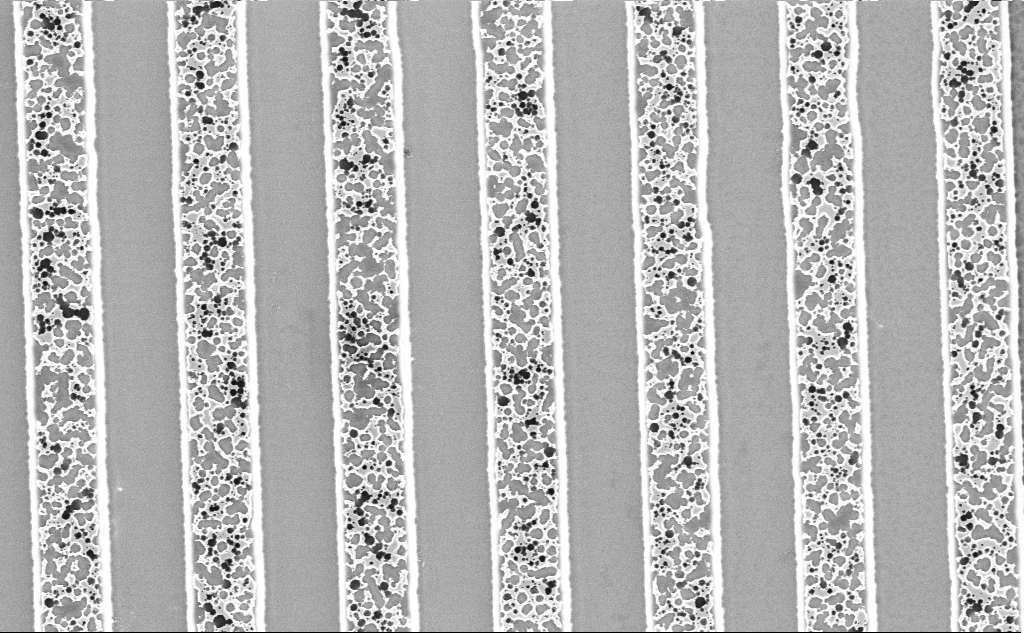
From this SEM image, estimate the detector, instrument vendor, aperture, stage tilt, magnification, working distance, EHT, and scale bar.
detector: InLens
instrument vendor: Zeiss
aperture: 30 µm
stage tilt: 0°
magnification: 13.97 K X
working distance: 7.9 mm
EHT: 3 kV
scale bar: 2000 nm